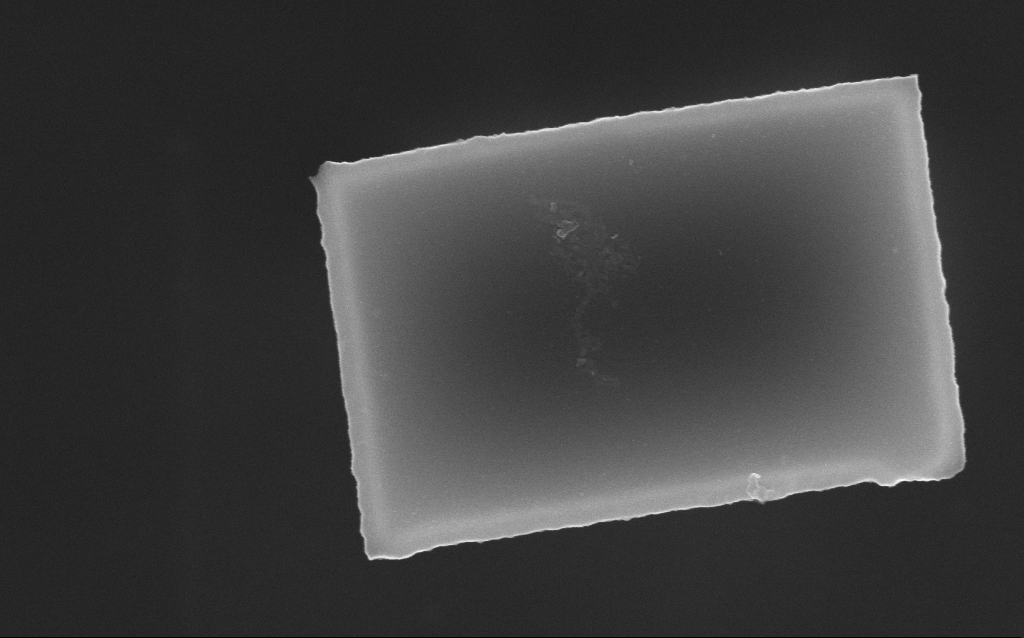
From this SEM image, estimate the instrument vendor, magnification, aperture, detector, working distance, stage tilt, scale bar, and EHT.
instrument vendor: Zeiss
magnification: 75.74 K X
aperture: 30 µm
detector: InLens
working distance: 8.4 mm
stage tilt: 0°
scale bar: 200 nm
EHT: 10 kV